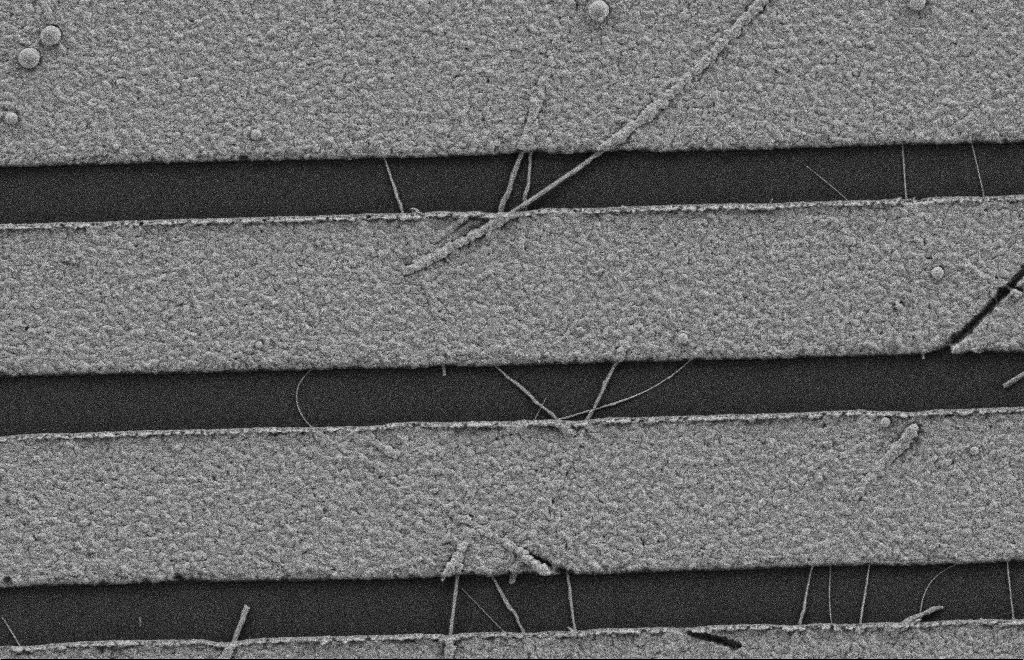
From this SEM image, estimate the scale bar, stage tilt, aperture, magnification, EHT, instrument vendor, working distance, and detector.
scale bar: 2000 nm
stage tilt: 0°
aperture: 20 µm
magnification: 19.35 K X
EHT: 2 kV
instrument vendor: Zeiss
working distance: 9 mm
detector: SE2